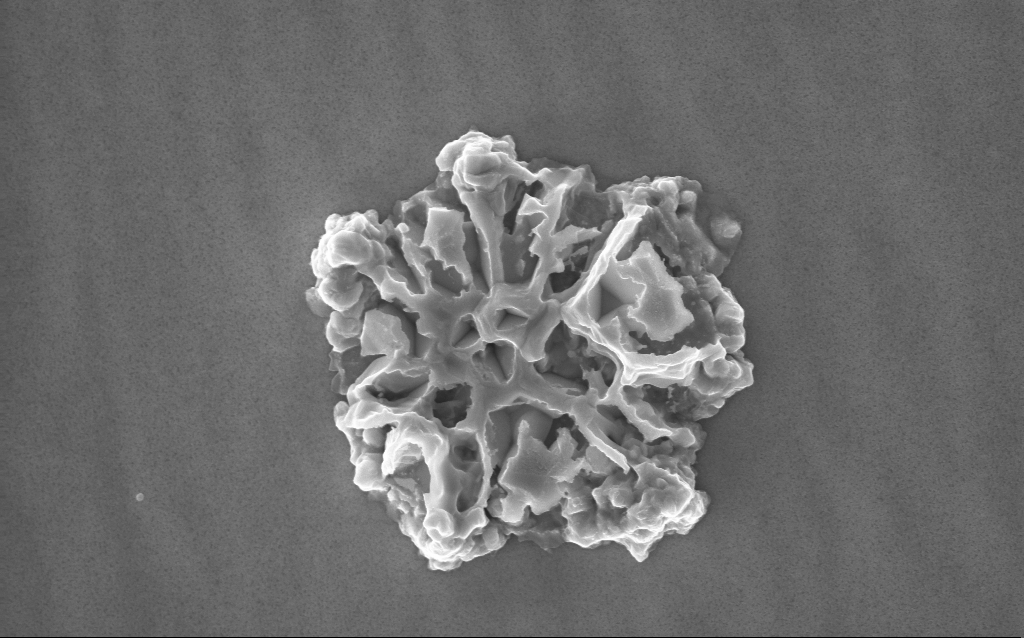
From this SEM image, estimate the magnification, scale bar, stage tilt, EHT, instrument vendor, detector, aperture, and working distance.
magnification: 52.56 K X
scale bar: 1000 nm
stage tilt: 0°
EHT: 10 kV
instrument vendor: Zeiss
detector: InLens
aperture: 30 µm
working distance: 2 mm